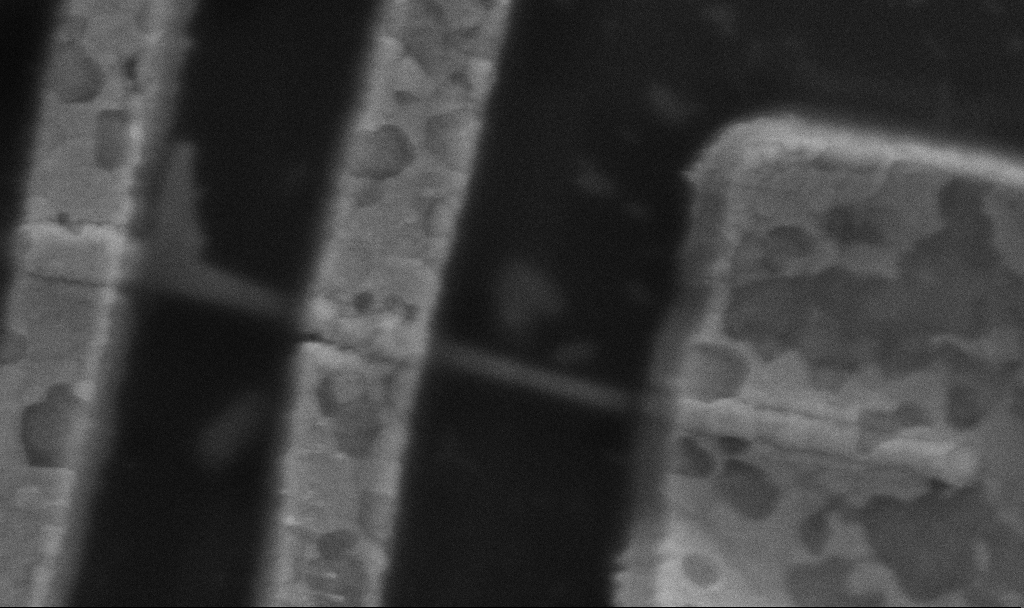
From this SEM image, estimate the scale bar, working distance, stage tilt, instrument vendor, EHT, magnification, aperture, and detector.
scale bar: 200 nm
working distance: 9.7 mm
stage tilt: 0°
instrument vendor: Zeiss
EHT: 5 kV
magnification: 100 K X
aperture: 30 µm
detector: SE2